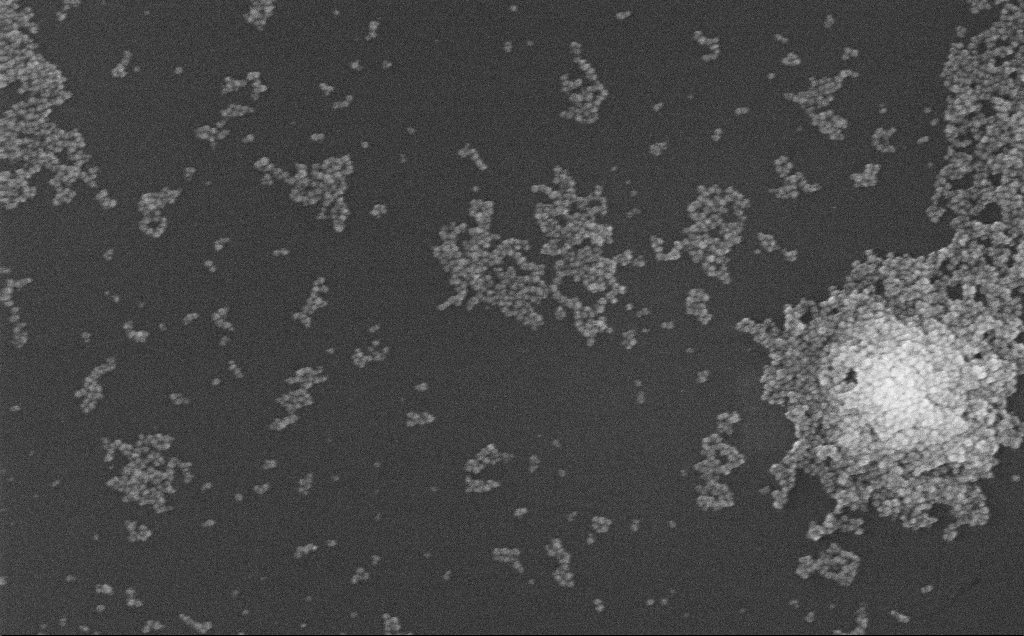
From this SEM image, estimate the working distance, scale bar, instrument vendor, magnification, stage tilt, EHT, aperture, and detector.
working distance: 3.7 mm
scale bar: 200 nm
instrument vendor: Zeiss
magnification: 100 K X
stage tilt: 0°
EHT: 10 kV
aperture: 30 µm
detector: InLens